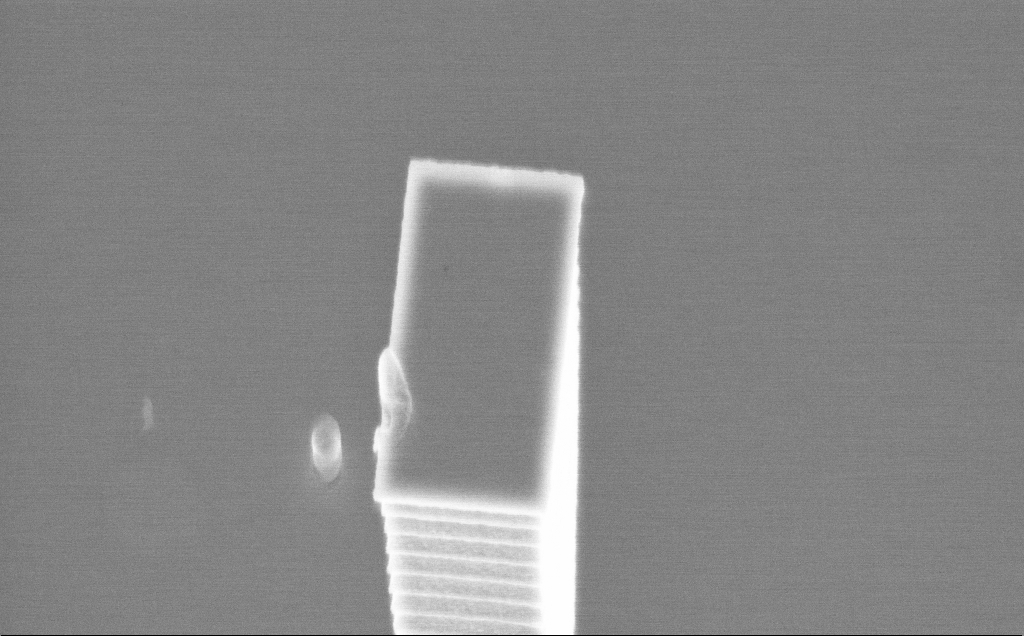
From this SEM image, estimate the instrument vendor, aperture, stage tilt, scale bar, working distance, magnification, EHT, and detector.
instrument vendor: Zeiss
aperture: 30 µm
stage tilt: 36.8°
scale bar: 2000 nm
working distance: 3 mm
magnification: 22.85 K X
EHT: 5 kV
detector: InLens